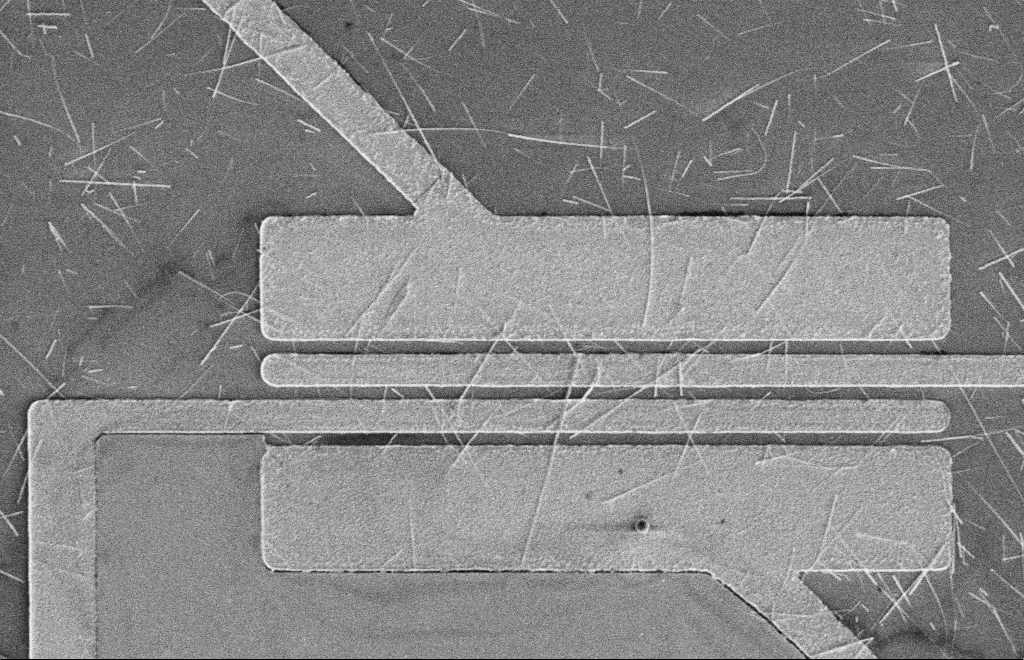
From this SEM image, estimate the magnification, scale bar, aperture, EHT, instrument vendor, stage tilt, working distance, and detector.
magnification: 4.18 K X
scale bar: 10000 nm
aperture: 10 µm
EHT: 5 kV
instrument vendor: Zeiss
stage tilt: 0°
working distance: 16 mm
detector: SE2